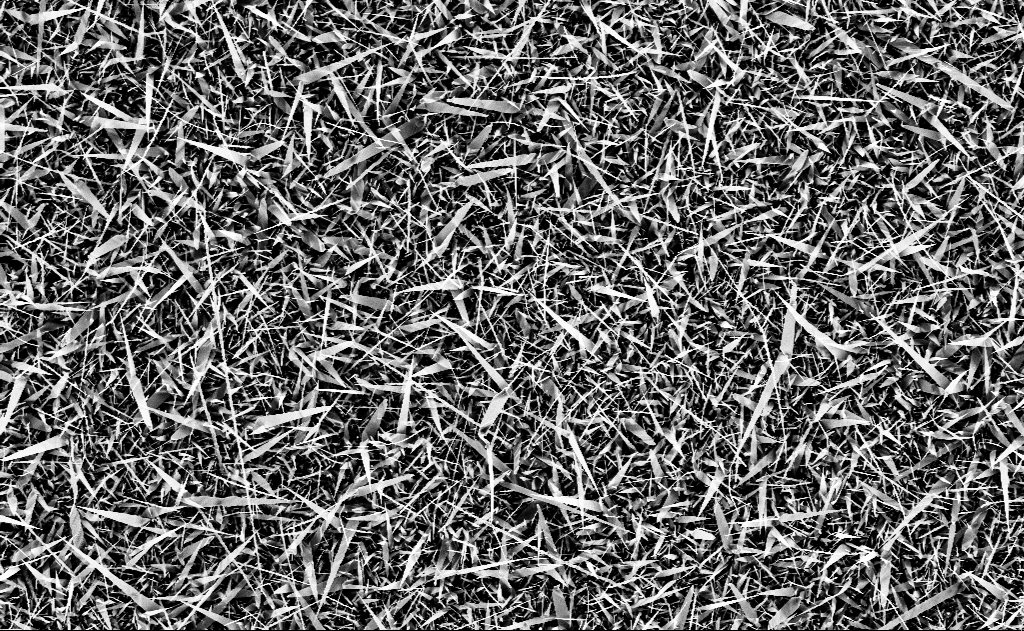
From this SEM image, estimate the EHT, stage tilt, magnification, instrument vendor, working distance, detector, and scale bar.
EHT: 10 kV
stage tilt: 0°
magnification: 3.3 K X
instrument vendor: Zeiss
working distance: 12 mm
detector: InLens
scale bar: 10000 nm